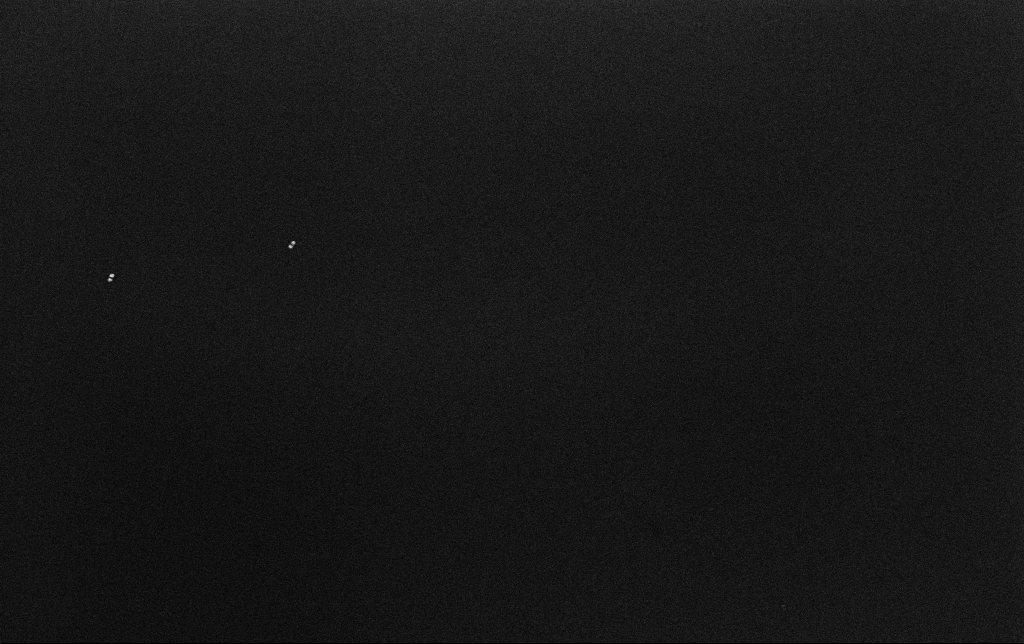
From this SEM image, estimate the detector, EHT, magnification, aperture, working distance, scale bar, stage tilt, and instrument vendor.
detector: InLens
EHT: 10 kV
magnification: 100 K X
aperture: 30 µm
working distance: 3.2 mm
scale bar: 200 nm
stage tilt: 0°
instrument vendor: Zeiss